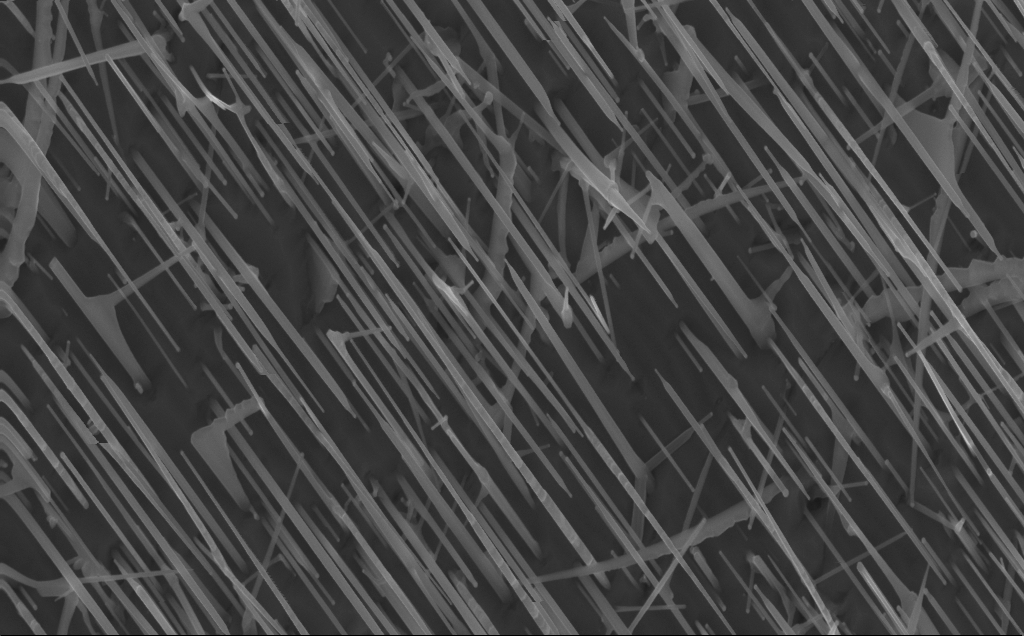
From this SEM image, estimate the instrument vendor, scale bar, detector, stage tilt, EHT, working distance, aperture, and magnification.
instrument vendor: Zeiss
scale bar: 1000 nm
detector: InLens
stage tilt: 0°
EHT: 10 kV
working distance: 4 mm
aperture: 30 µm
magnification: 40 K X